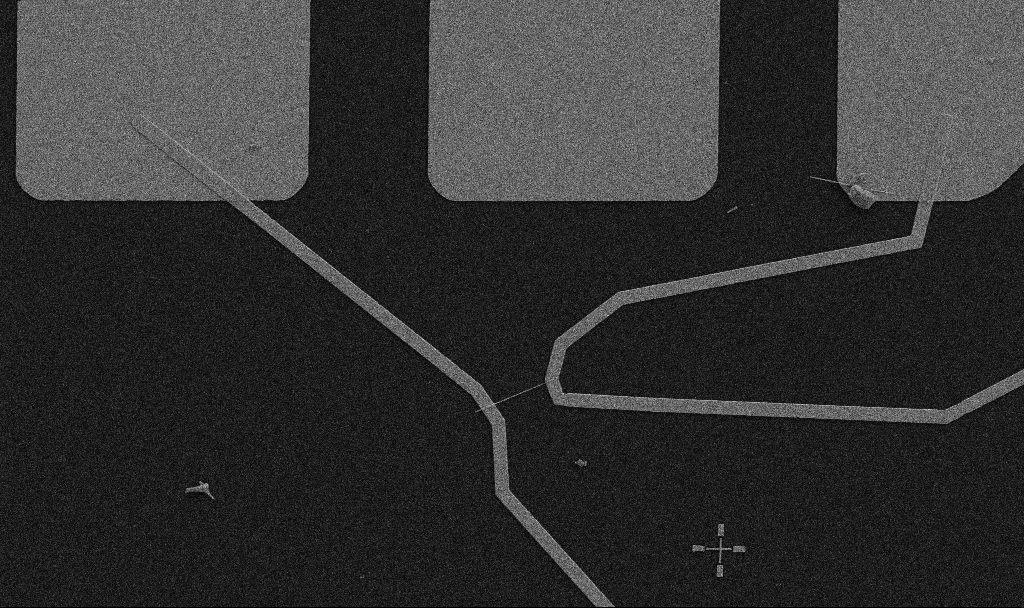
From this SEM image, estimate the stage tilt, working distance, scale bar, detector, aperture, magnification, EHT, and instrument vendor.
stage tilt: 0°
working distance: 10.7 mm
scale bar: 10000 nm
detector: SE2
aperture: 30 µm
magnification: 5 K X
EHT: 5 kV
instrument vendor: Zeiss